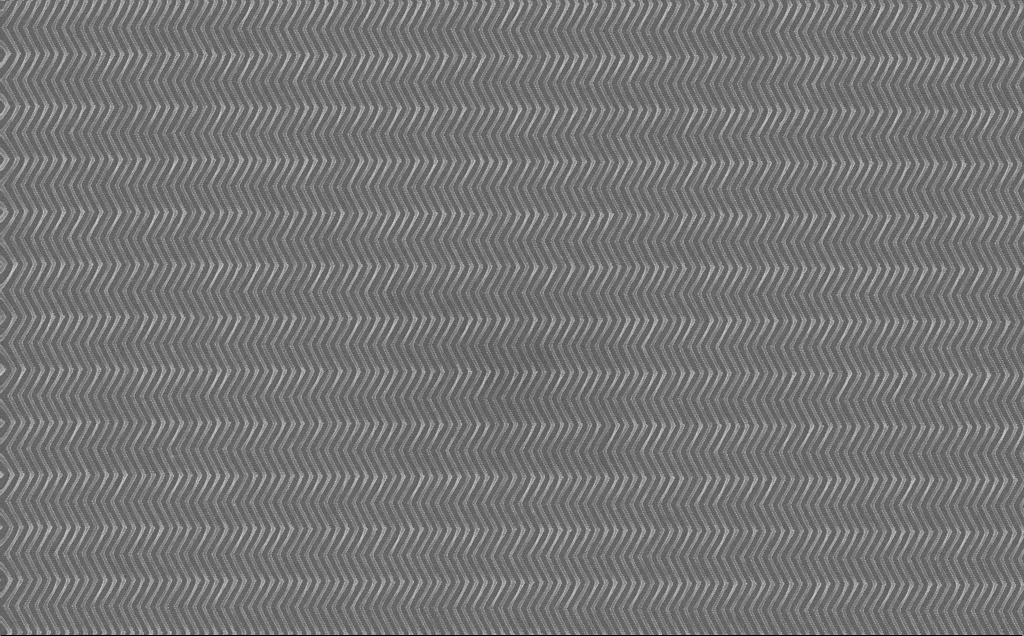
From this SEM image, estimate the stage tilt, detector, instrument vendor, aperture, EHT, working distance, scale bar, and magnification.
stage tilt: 0°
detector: InLens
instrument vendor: Zeiss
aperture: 30 µm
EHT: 10 kV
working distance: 7 mm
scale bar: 2000 nm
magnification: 9.75 K X